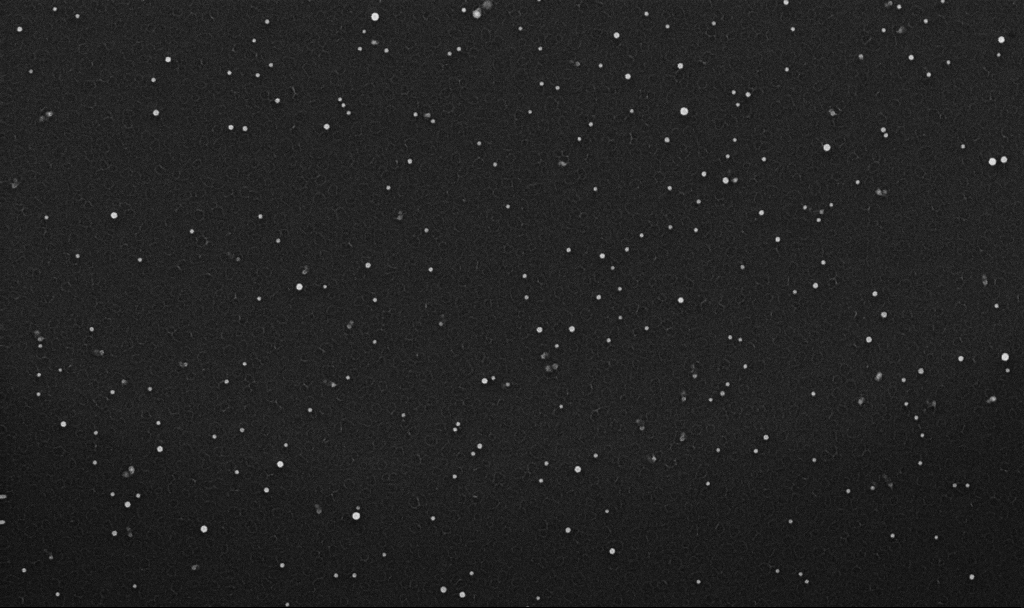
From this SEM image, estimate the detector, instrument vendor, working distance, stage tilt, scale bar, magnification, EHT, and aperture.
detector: InLens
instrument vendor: Zeiss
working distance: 3.3 mm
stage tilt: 0°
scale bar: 1000 nm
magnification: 70 K X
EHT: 10 kV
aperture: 30 µm